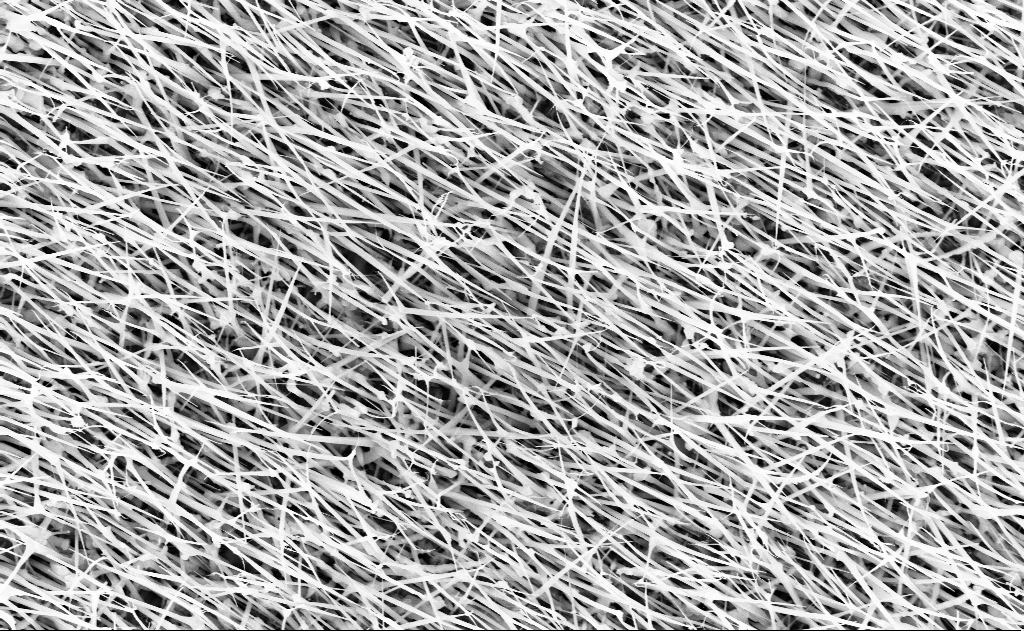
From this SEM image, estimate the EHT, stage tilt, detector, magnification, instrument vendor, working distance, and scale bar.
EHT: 10 kV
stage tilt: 0°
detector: InLens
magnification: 20 K X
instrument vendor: Zeiss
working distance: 13 mm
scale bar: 1000 nm